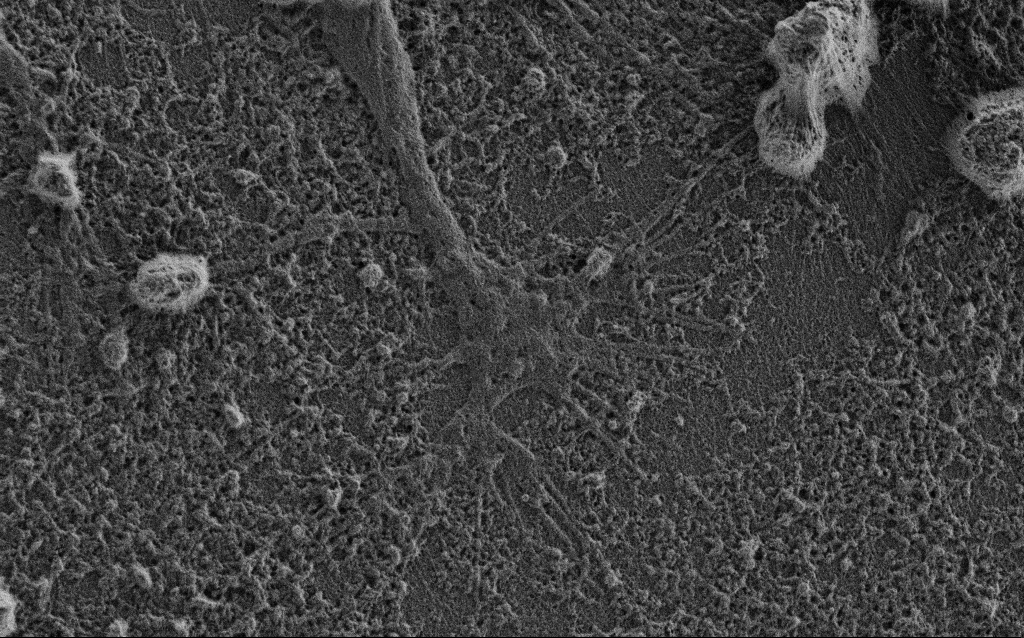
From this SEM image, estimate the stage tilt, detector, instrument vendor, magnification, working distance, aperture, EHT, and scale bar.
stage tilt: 0°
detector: SE2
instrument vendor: Zeiss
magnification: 12.5 K X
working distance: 3.4 mm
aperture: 30 µm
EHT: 0.9 kV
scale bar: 2000 nm